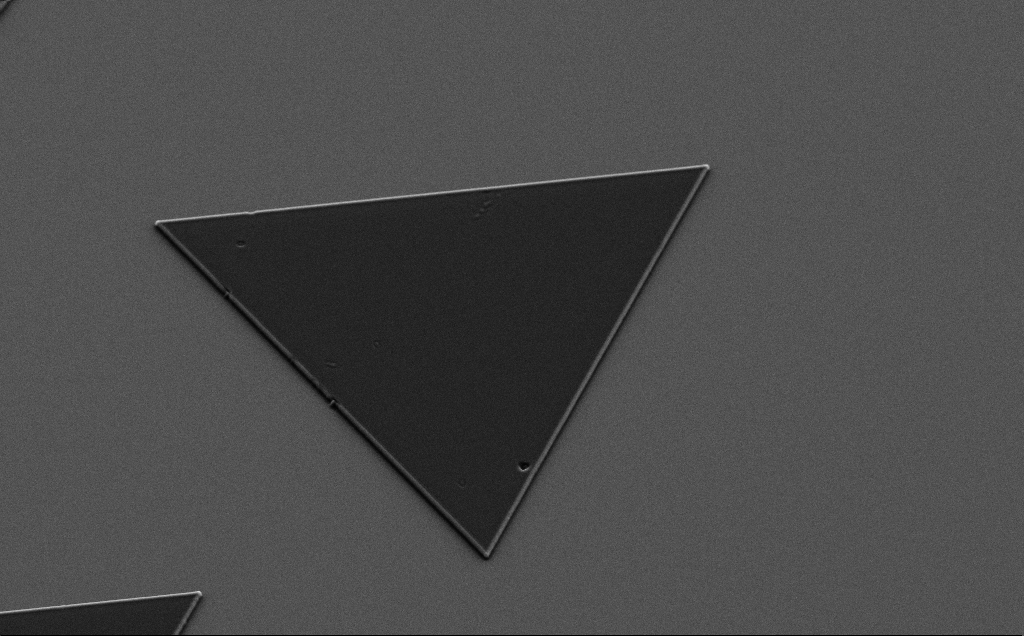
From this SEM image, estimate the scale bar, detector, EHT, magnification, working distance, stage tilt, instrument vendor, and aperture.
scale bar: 20000 nm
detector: SE2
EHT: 7 kV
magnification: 1.8 K X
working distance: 6 mm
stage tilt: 35°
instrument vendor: Zeiss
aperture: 30 µm